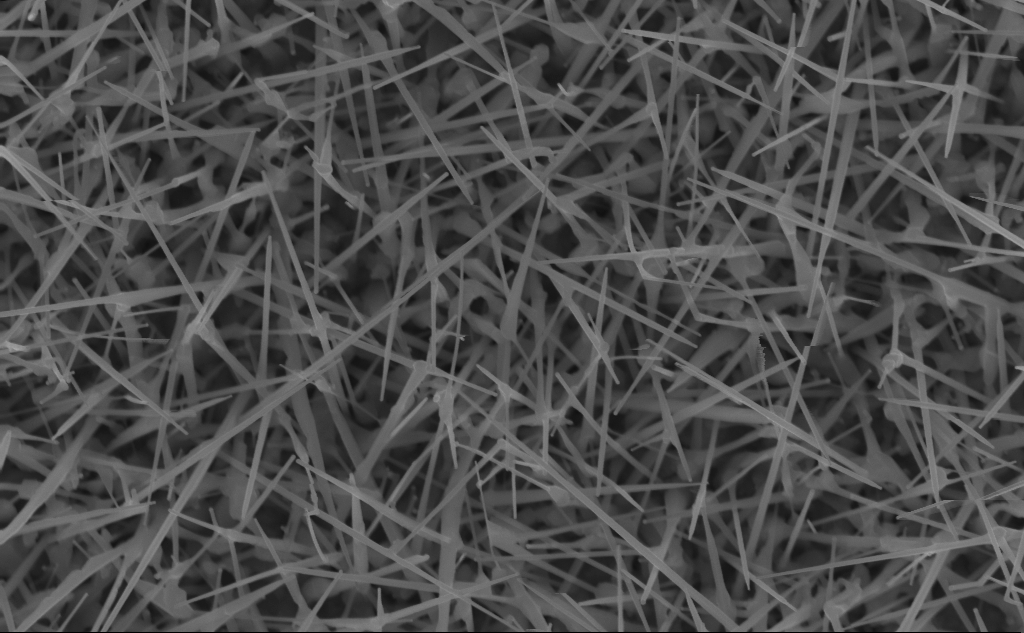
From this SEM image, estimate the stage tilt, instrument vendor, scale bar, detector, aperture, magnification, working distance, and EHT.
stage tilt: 0°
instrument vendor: Zeiss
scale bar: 1000 nm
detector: InLens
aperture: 30 µm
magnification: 40 K X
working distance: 5 mm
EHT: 10 kV